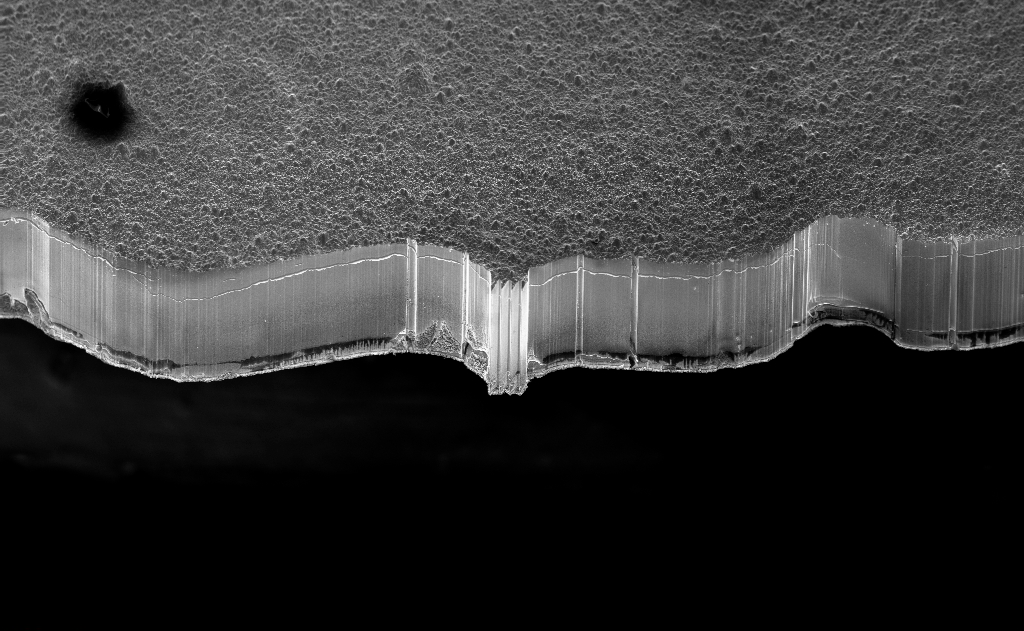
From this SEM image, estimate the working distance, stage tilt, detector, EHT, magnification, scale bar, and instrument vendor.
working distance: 4 mm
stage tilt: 45°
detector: InLens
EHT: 10 kV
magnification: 0.327 K X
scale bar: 100000 nm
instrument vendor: Zeiss